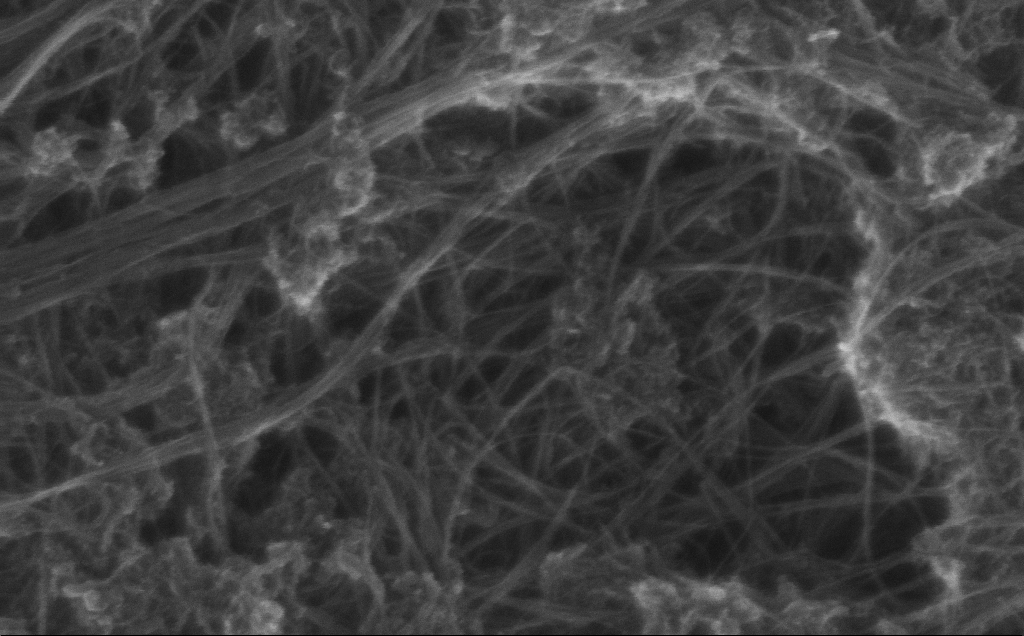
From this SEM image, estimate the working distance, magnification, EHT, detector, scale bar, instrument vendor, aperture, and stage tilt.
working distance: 3 mm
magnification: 225.97 K X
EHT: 10 kV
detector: InLens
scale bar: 200 nm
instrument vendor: Zeiss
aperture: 30 µm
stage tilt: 0°